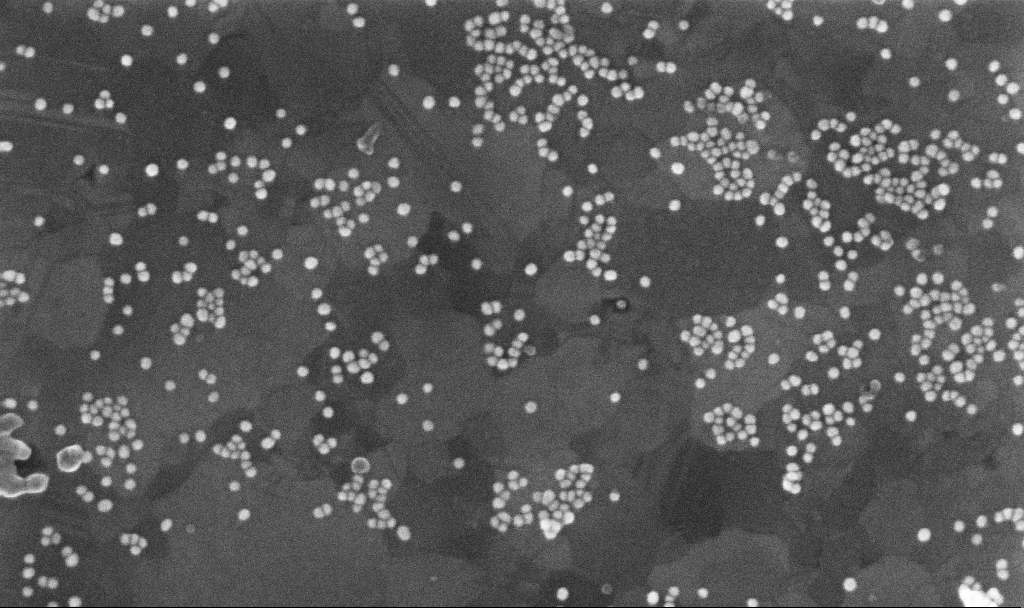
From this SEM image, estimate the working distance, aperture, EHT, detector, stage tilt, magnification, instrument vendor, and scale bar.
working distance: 3.7 mm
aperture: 30 µm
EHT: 10 kV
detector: InLens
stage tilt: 0°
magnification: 200 K X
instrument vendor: Zeiss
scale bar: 200 nm